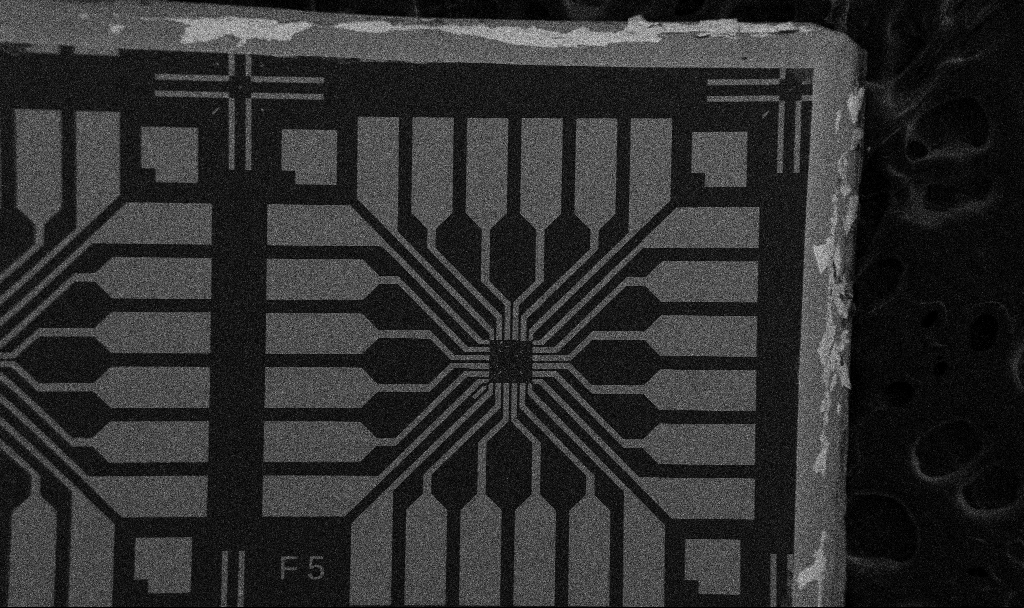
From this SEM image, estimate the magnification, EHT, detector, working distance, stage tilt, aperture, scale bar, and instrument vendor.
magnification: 0.1 K X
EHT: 5 kV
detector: SE2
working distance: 10.7 mm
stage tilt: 0°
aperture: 30 µm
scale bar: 200000 nm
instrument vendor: Zeiss